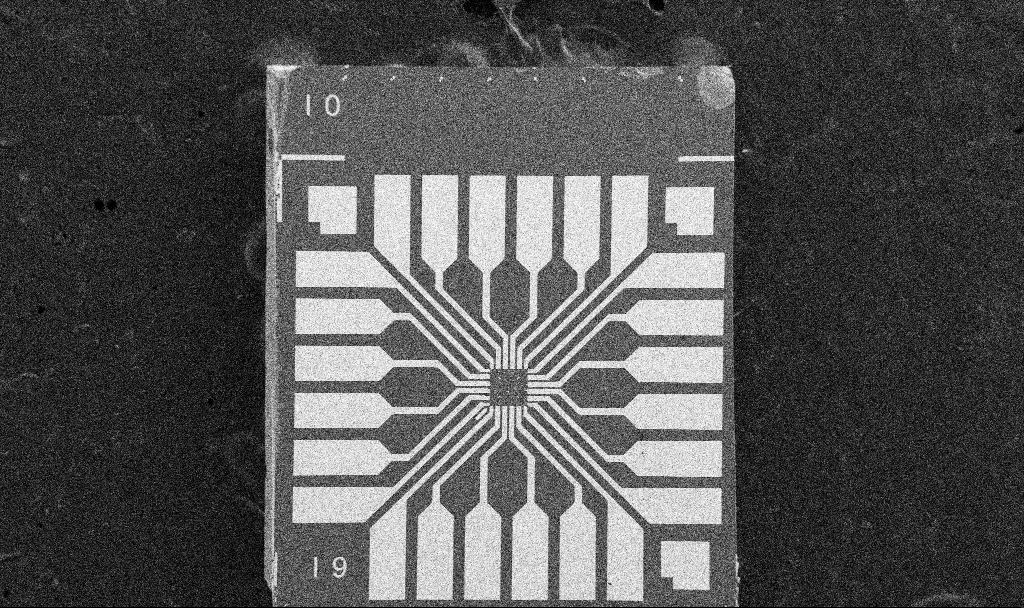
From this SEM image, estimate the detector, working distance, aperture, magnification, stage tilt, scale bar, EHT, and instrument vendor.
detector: SE2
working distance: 10.5 mm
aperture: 30 µm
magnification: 0.089 K X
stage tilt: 0°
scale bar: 200000 nm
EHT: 5 kV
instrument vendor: Zeiss